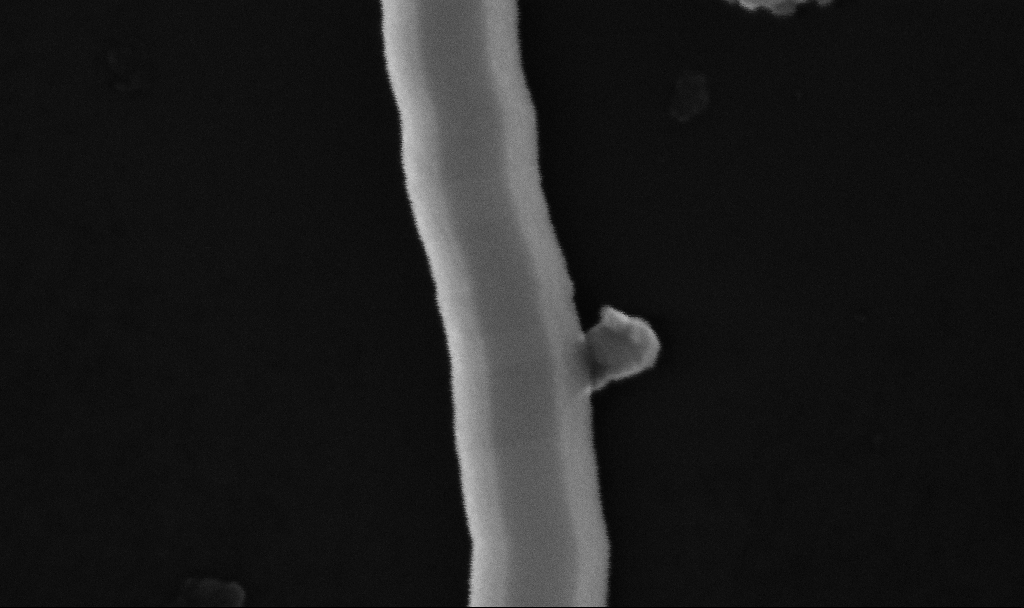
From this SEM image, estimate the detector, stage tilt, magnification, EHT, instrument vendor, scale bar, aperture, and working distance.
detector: InLens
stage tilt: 0°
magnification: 456.3 K X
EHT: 10 kV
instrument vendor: Zeiss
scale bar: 100 nm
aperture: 30 µm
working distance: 6.7 mm